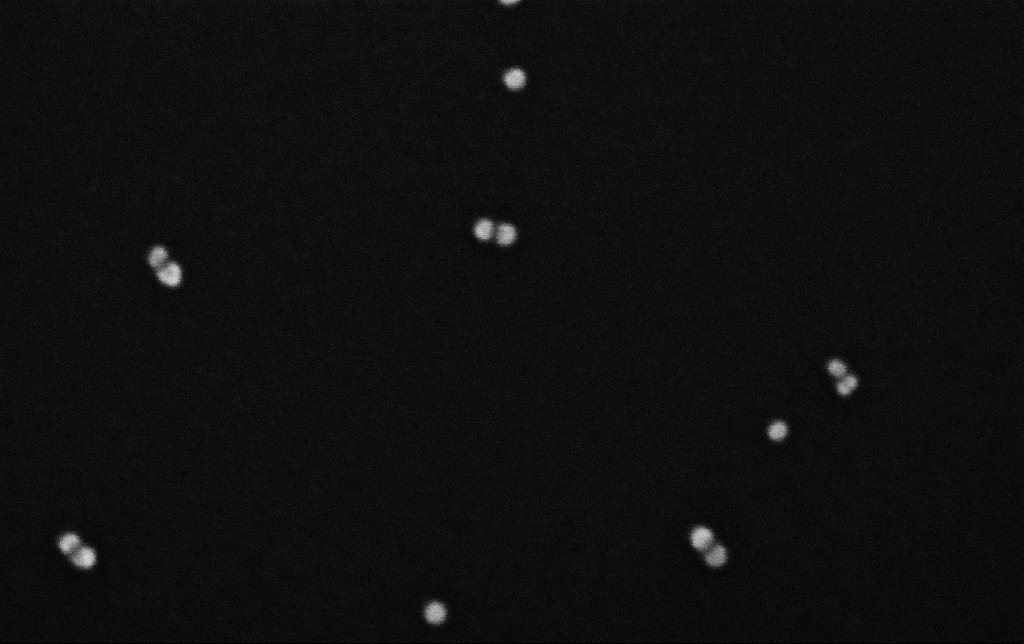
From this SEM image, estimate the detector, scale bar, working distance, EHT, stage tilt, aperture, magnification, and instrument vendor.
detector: InLens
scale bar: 100 nm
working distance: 3.1 mm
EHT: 10 kV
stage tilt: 0°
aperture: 30 µm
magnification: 573.58 K X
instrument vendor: Zeiss